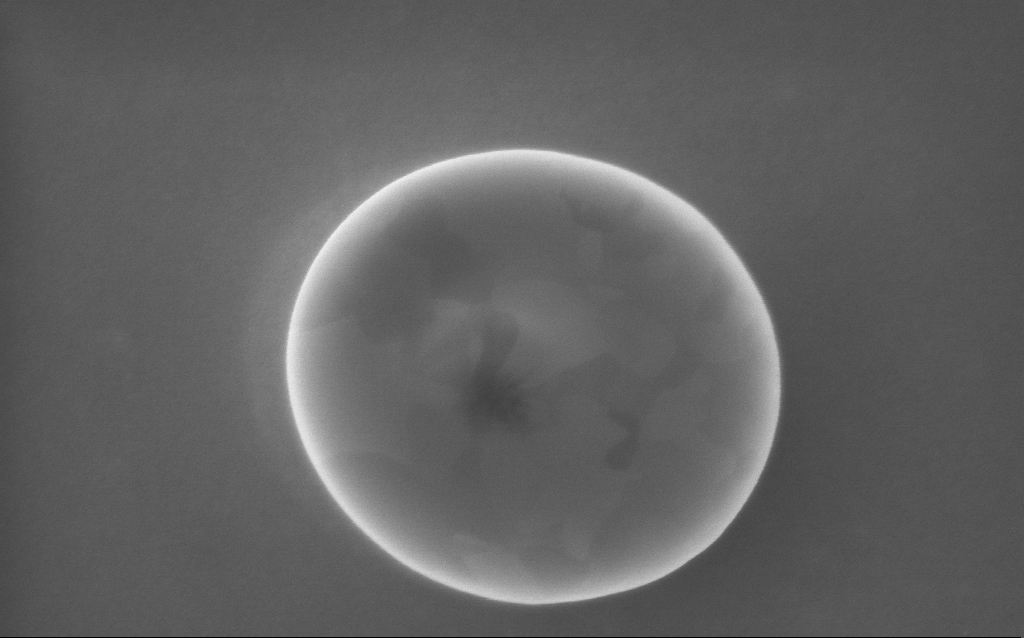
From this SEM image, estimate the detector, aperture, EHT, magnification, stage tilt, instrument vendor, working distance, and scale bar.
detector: InLens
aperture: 30 µm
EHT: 5 kV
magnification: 105.11 K X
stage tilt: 0°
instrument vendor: Zeiss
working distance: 2 mm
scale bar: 200 nm